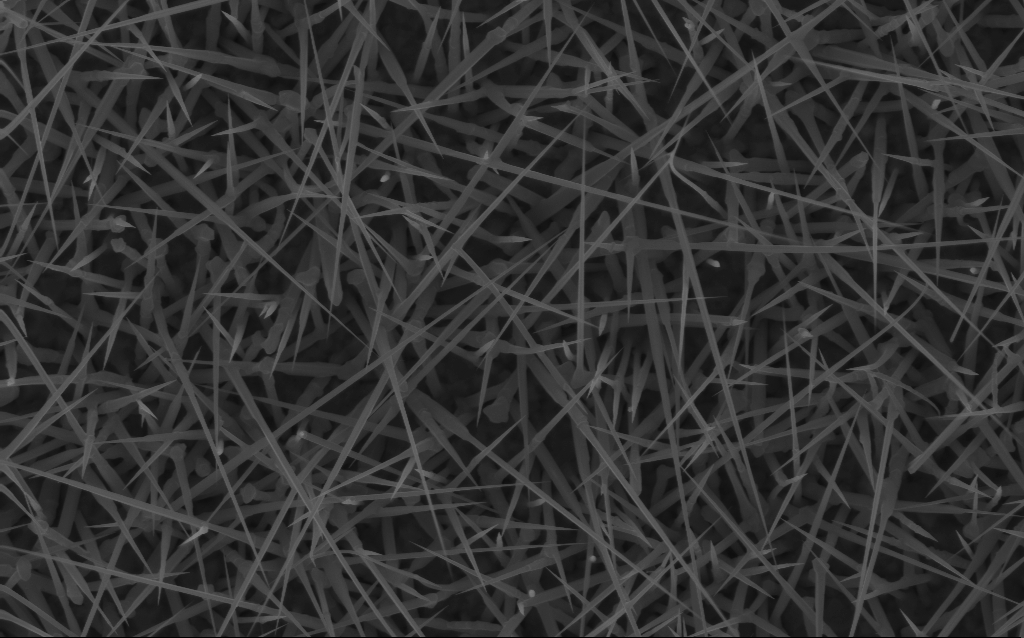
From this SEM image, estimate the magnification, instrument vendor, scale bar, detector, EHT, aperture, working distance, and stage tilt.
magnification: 20 K X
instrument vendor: Zeiss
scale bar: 1000 nm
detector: InLens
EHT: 10 kV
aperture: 30 µm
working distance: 7 mm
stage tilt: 0°